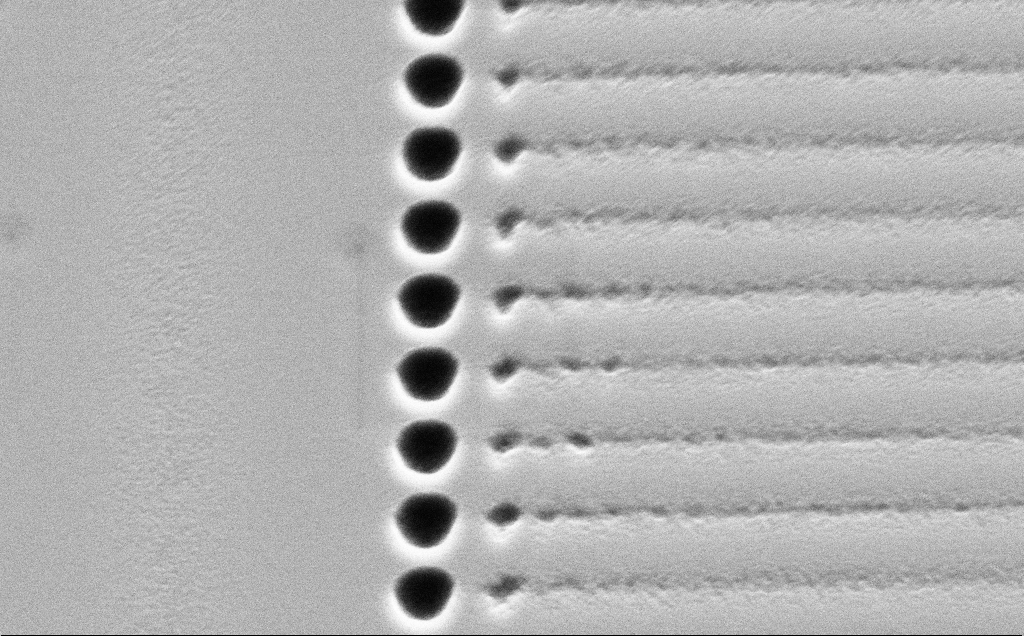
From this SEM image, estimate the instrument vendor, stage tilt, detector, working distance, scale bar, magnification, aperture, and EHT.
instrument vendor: Zeiss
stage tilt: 45°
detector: SE2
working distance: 9 mm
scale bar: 2000 nm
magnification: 14.07 K X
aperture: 30 µm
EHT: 5 kV